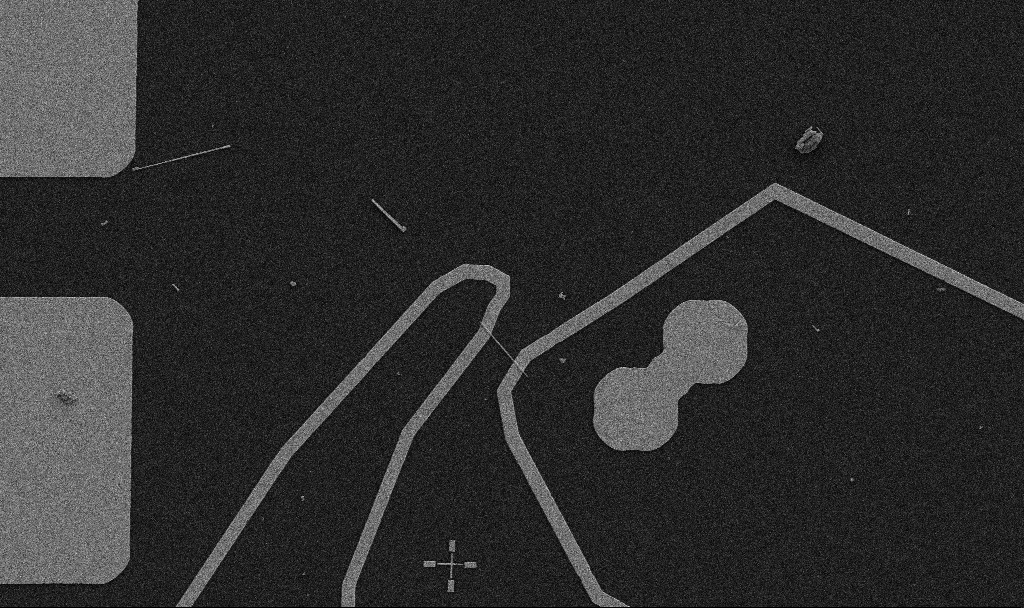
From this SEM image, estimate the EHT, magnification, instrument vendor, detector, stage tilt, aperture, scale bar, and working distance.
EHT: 5 kV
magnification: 5 K X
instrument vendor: Zeiss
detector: SE2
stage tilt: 0°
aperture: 30 µm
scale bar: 10000 nm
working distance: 10.7 mm